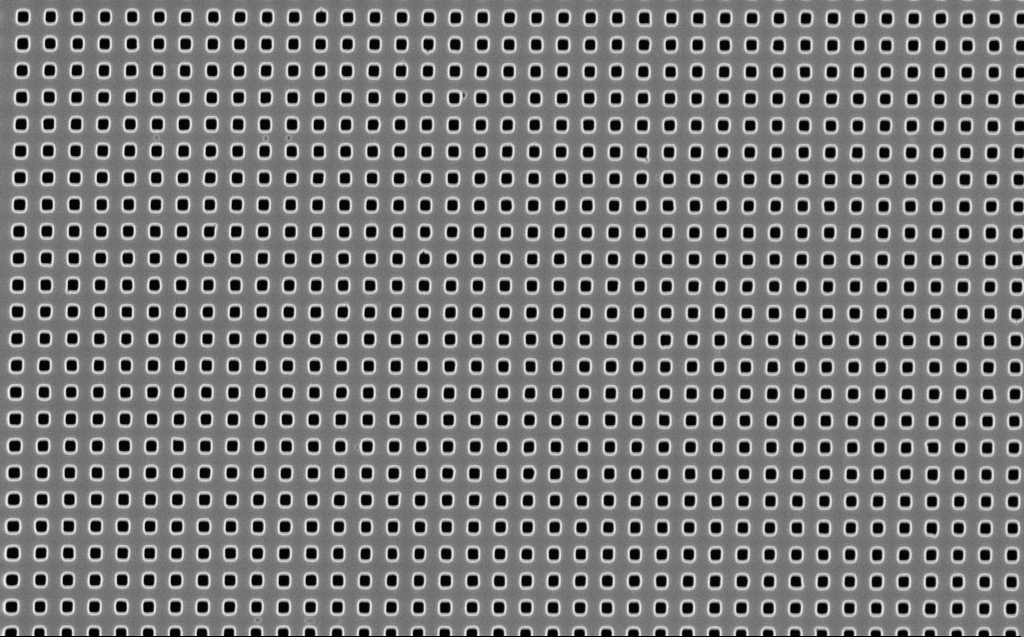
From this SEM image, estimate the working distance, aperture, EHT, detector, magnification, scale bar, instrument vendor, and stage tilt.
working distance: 6 mm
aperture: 30 µm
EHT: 10 kV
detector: InLens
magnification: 20 K X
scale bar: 2000 nm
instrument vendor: Zeiss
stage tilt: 0°